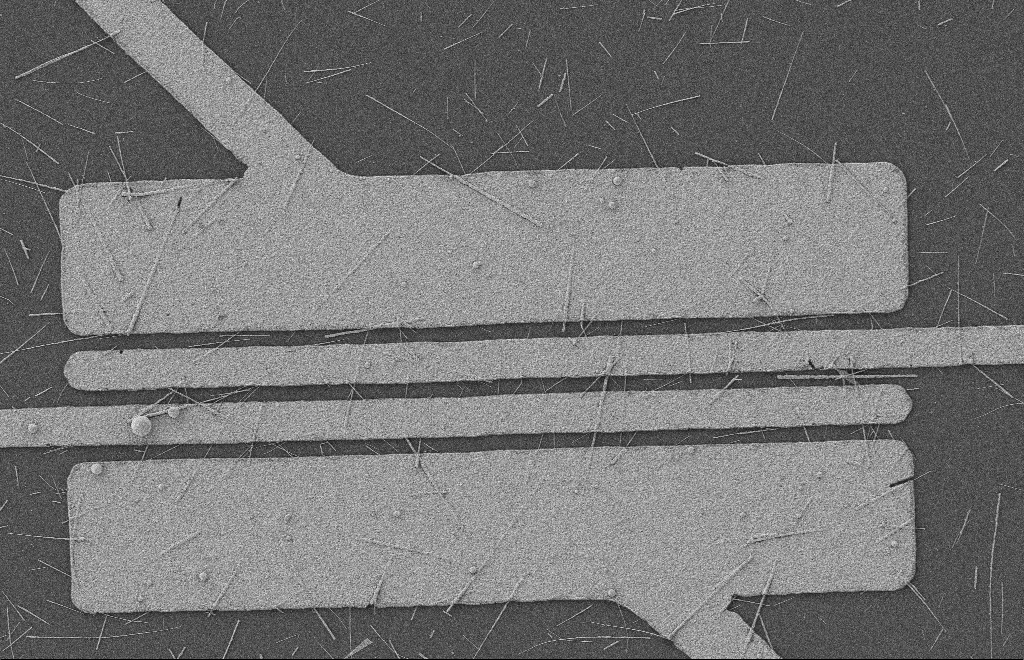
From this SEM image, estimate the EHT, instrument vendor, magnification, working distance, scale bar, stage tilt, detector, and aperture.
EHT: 2 kV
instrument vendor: Zeiss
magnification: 5.08 K X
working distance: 8 mm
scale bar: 2000 nm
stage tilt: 0°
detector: SE2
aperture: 20 µm